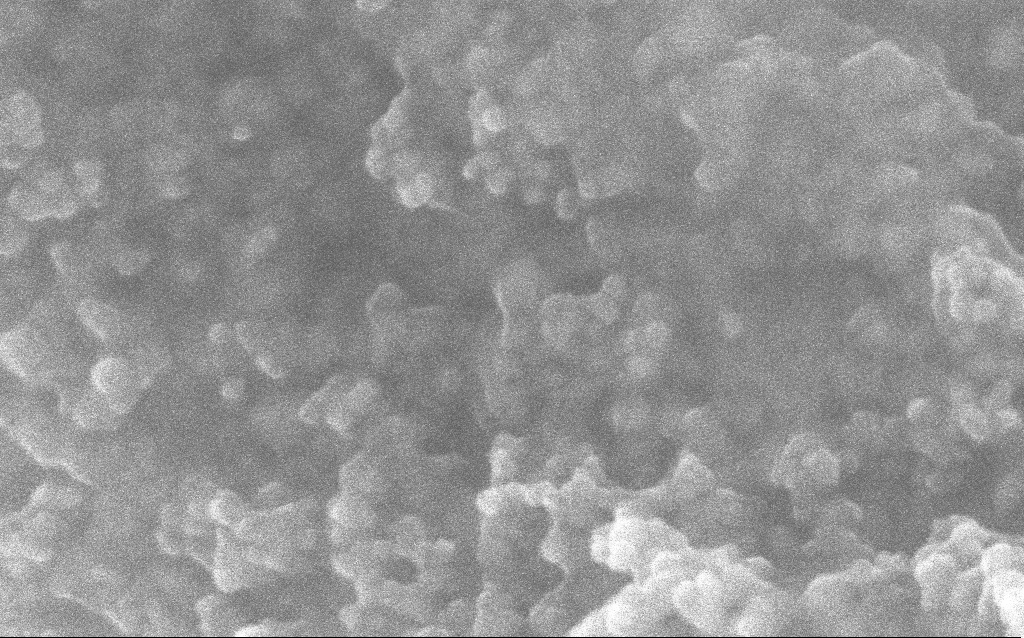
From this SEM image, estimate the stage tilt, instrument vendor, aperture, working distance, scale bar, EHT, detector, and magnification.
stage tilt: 0°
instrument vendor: Zeiss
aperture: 30 µm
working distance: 2.8 mm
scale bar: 100 nm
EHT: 10 kV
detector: InLens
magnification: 348.1 K X